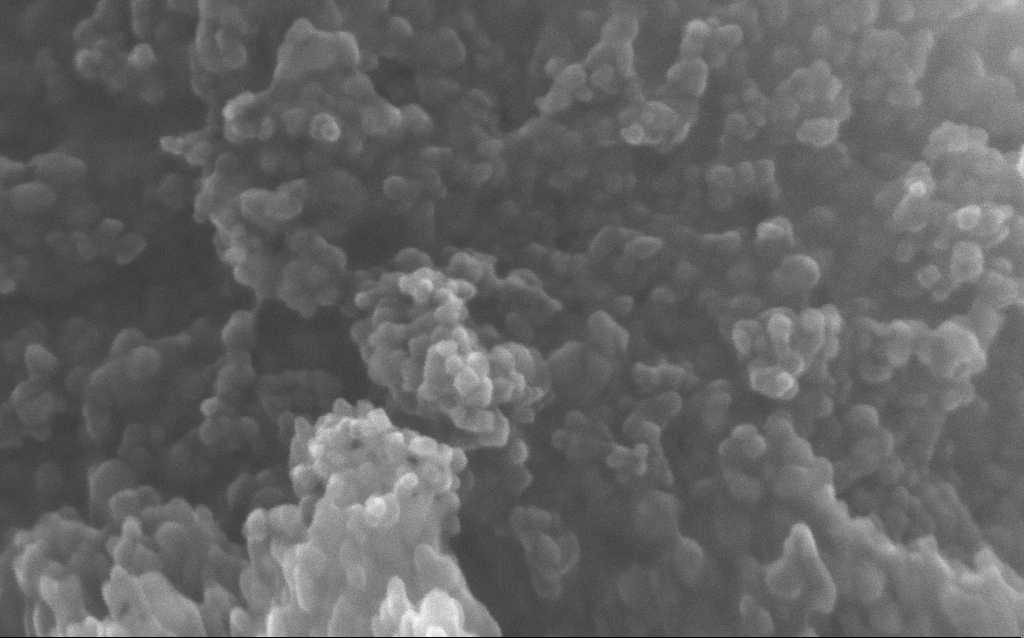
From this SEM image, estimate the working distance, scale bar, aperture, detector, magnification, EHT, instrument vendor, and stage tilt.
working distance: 2.7 mm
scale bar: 100 nm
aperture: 30 µm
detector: InLens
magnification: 348.1 K X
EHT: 10 kV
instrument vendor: Zeiss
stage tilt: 0°